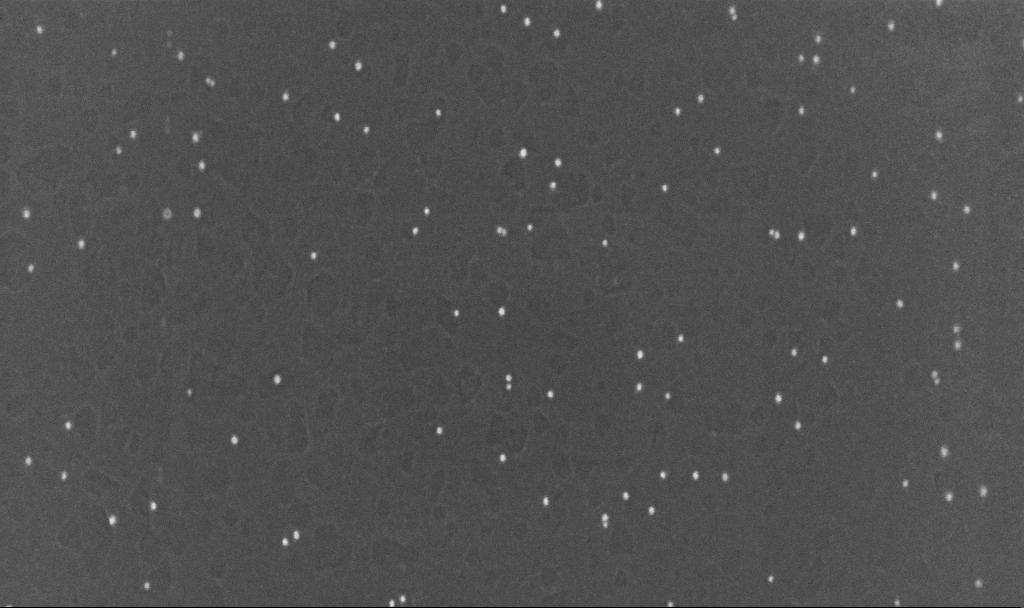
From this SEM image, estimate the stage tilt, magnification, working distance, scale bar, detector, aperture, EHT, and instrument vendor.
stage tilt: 0°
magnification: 200 K X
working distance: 3.1 mm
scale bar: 100 nm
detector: InLens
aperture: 30 µm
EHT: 10 kV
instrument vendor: Zeiss